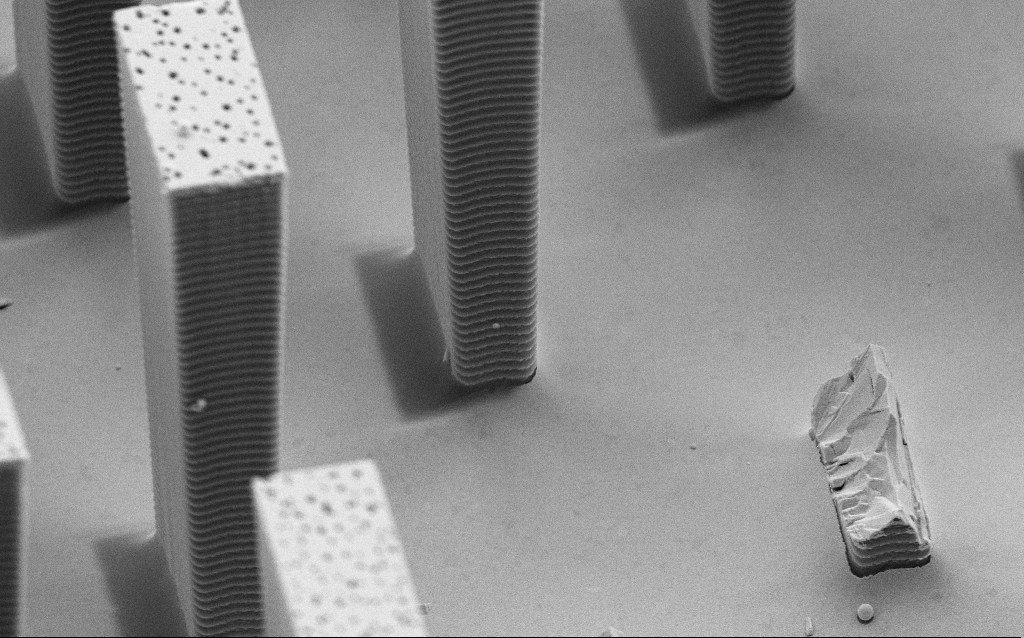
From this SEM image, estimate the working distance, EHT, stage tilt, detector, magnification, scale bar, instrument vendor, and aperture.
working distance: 8 mm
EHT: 5 kV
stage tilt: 45°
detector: SE2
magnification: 12.24 K X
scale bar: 2000 nm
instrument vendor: Zeiss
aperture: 30 µm